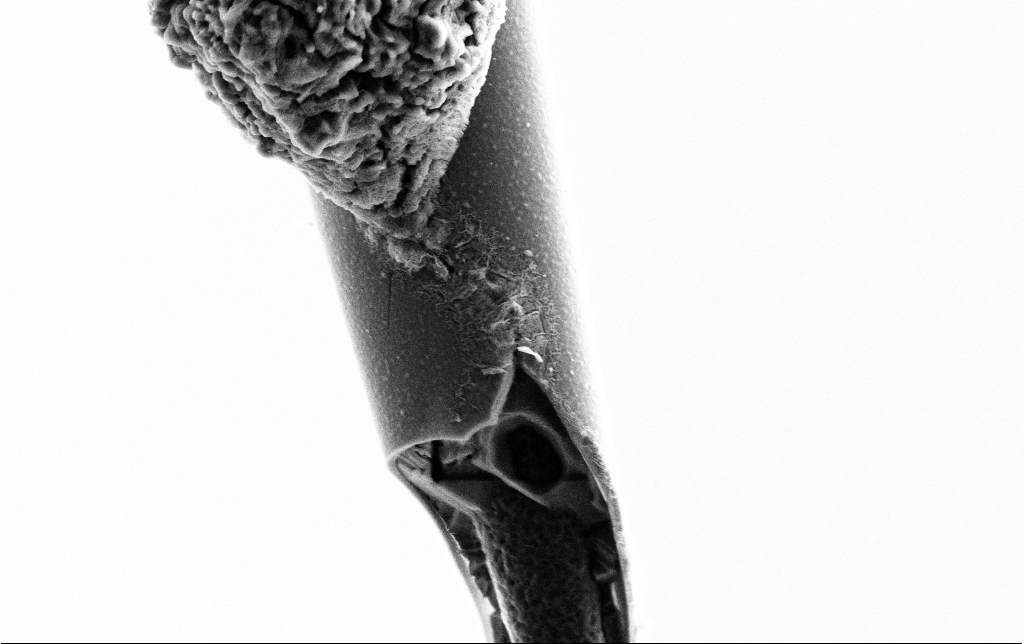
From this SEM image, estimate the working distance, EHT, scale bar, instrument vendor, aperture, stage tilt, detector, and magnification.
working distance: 6.5 mm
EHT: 3 kV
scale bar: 2000 nm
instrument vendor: Zeiss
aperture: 30 µm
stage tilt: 0°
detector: SE2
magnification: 20 K X